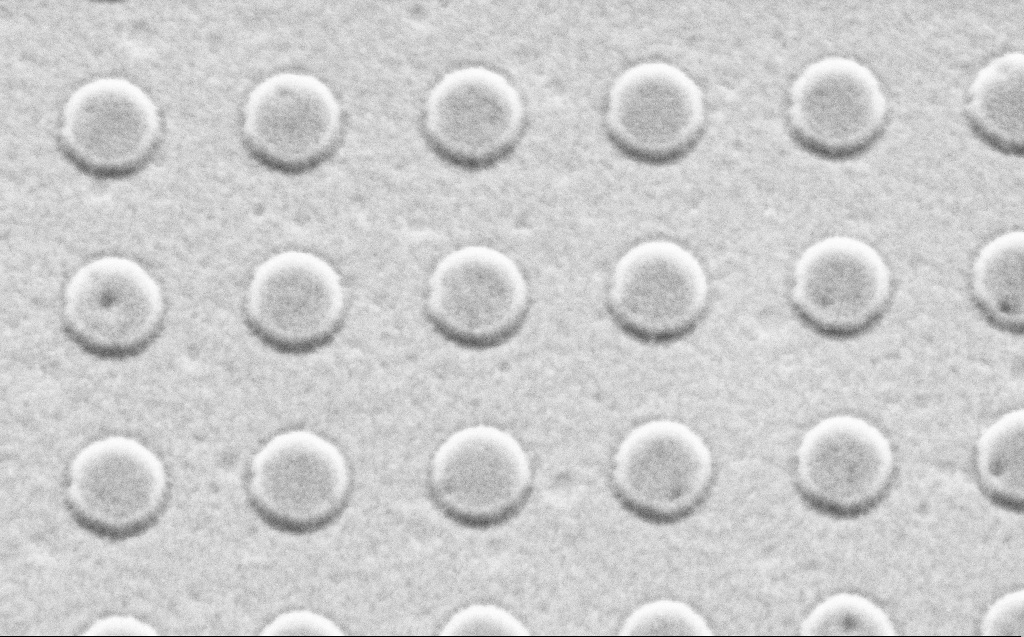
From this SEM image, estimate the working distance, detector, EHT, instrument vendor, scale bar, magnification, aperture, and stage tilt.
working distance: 4 mm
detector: SE2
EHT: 2.5 kV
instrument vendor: Zeiss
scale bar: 1000 nm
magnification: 56.14 K X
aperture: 30 µm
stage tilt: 30°